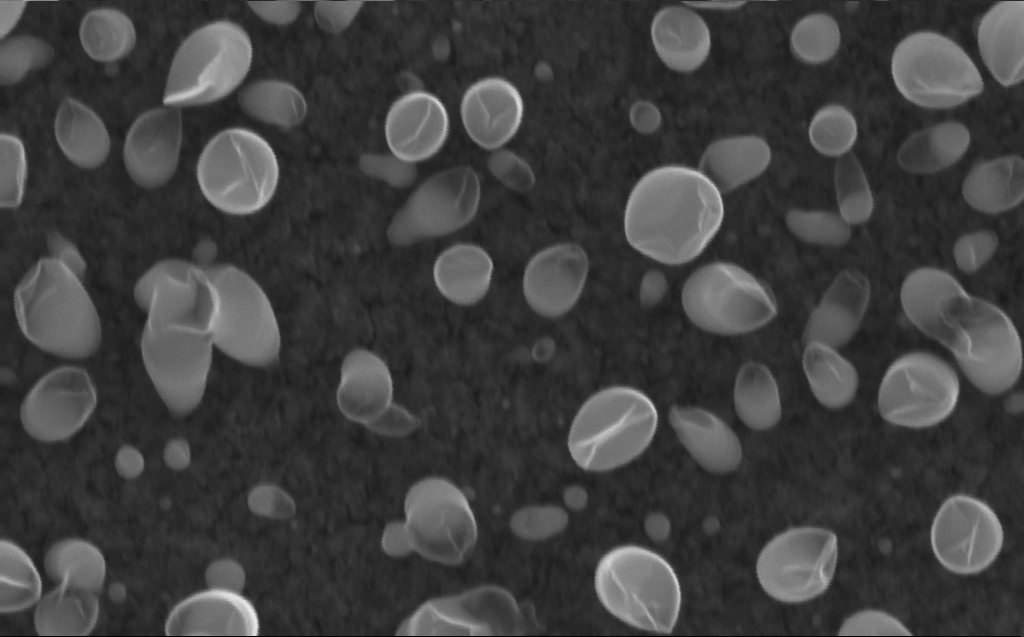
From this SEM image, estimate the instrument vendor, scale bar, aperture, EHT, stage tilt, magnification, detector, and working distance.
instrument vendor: Zeiss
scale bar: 100 nm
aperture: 30 µm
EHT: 10 kV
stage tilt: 0°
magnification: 200 K X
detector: InLens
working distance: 3 mm